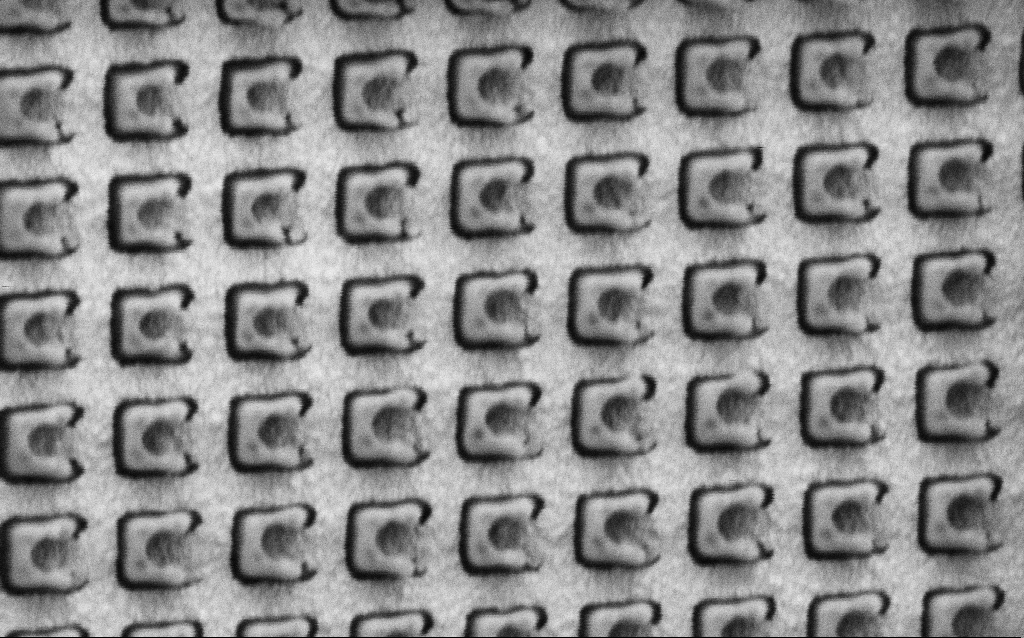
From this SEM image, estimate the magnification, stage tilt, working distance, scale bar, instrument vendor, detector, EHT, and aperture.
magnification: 90.15 K X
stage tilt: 0°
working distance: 8 mm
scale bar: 200 nm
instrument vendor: Zeiss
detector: SE2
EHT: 1.5 kV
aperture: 30 µm